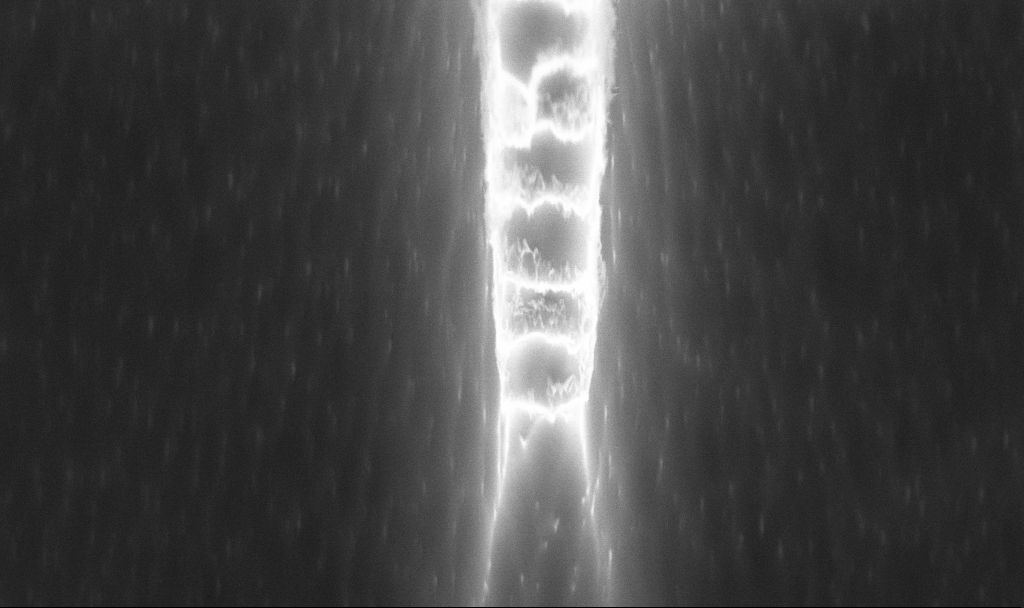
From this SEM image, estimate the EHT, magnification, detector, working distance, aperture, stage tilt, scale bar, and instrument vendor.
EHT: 5 kV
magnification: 55.07 K X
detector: InLens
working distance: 4.7 mm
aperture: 30 µm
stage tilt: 20°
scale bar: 1000 nm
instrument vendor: Zeiss